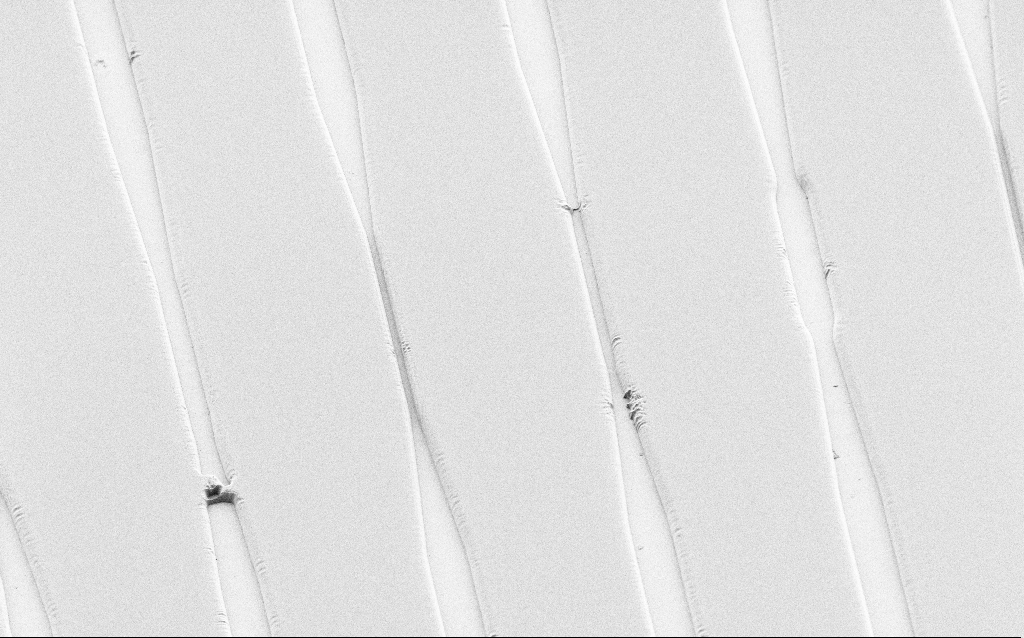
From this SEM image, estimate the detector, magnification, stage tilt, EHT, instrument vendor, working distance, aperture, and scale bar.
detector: SE2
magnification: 0.721 K X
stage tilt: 36°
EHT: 1 kV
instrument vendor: Zeiss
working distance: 6 mm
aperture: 30 µm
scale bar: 100000 nm